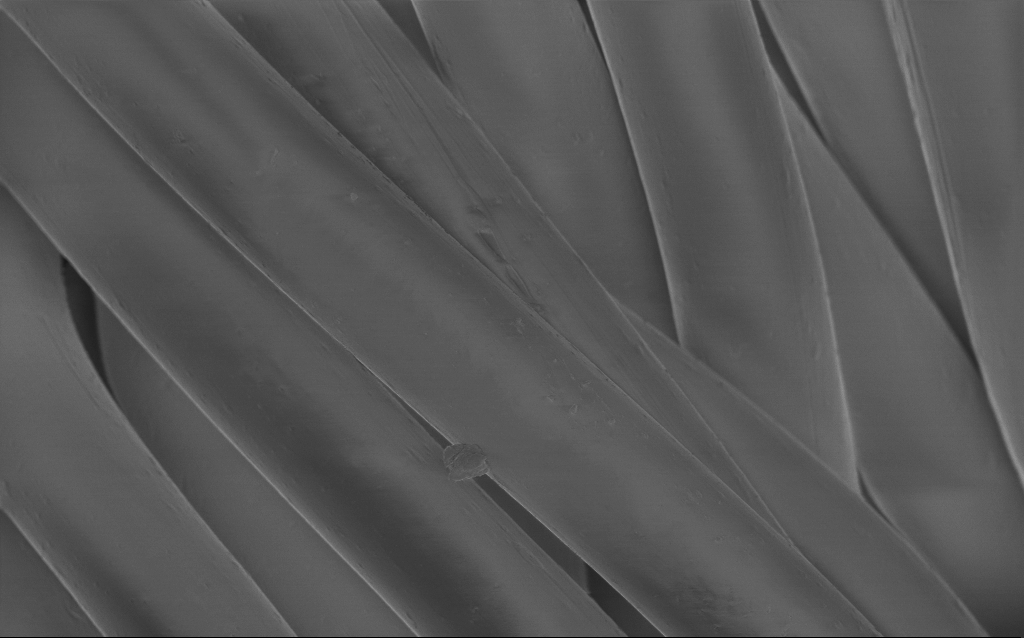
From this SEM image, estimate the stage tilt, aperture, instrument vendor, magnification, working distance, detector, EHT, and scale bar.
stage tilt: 0°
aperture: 30 µm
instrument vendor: Zeiss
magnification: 2.4 K X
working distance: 4 mm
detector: InLens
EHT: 1 kV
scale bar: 10000 nm